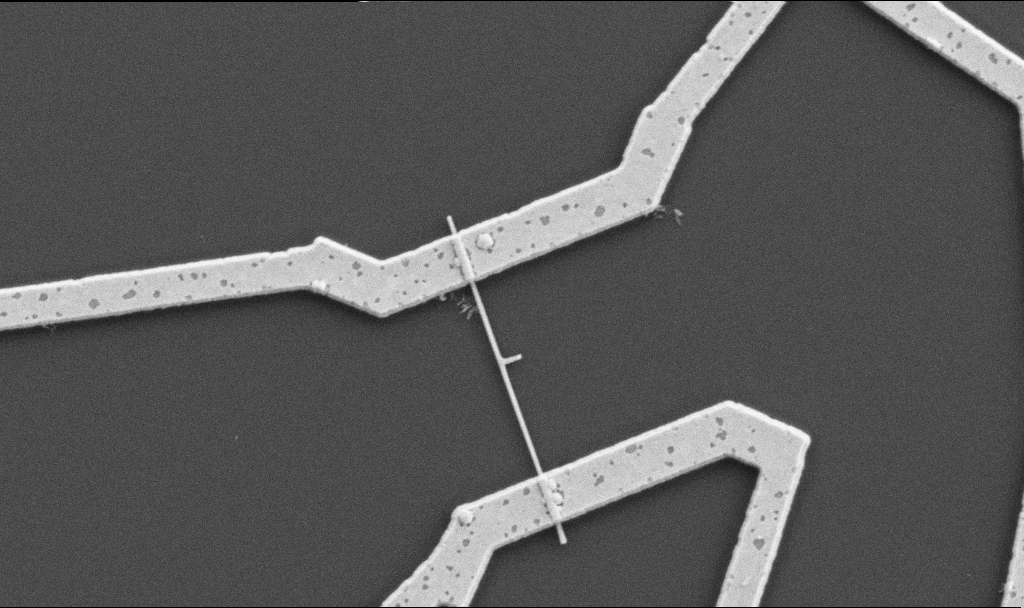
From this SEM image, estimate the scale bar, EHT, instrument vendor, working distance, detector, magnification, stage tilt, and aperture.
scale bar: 1000 nm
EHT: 5 kV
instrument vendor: Zeiss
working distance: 10.7 mm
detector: SE2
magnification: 20 K X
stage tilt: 0°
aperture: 30 µm